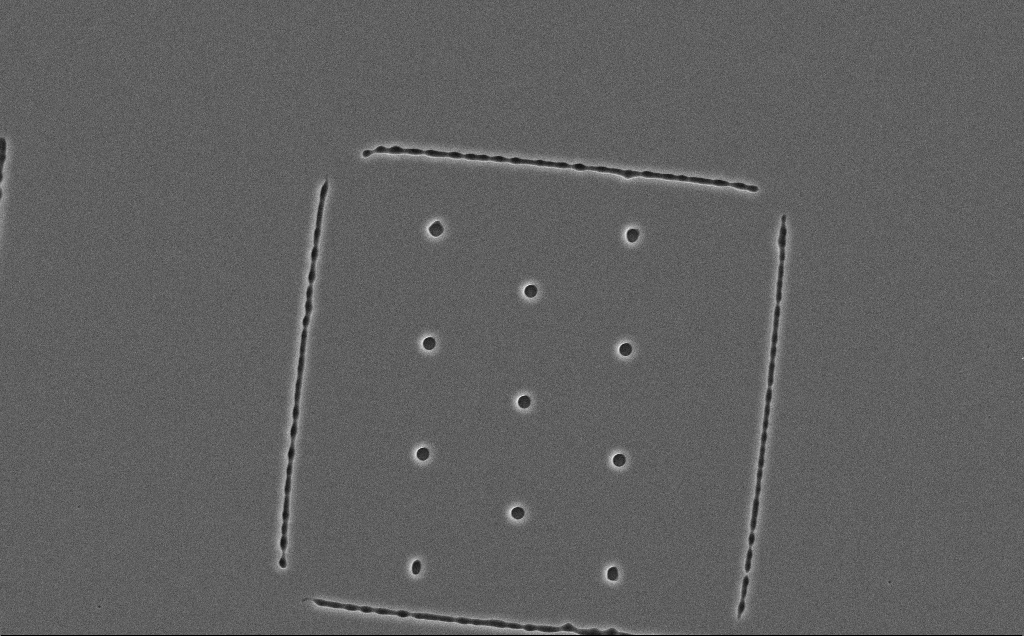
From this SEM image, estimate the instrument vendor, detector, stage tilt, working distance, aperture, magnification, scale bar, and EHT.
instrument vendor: Zeiss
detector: SE2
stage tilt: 0°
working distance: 16 mm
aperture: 30 µm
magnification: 2.03 K X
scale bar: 20000 nm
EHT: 10 kV